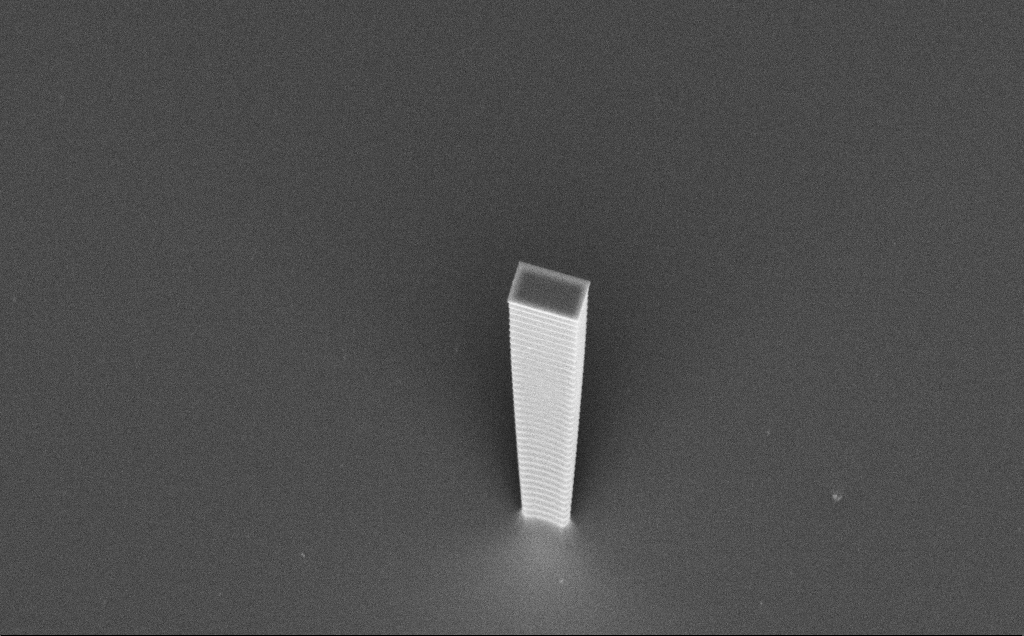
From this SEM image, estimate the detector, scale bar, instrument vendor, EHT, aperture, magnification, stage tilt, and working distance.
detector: InLens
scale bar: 10000 nm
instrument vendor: Zeiss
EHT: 7.5 kV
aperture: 30 µm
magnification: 5.67 K X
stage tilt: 45°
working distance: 8 mm